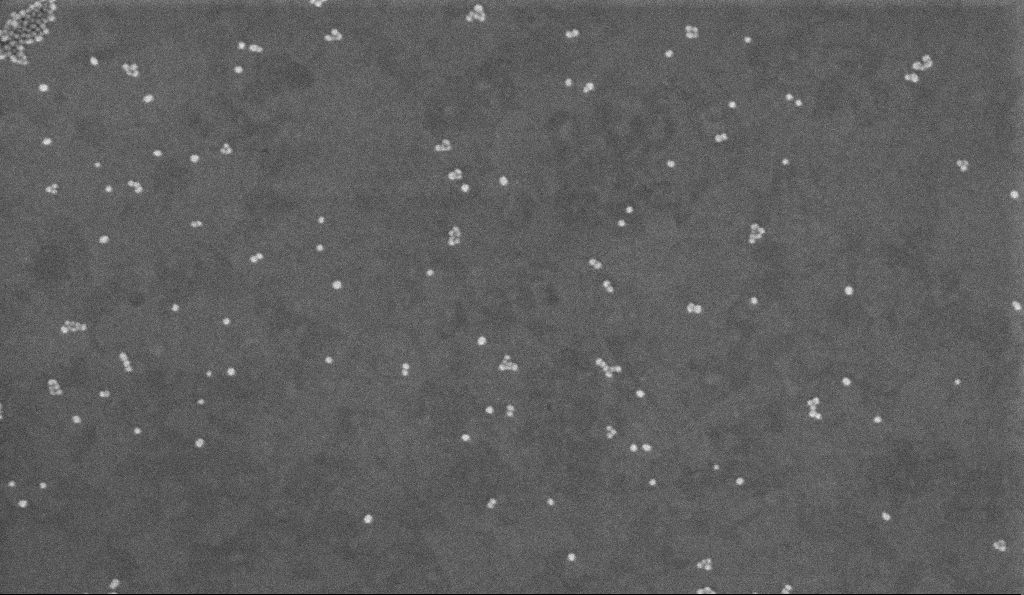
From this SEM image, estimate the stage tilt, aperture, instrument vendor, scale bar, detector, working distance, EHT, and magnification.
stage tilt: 0°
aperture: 30 µm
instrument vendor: Zeiss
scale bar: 200 nm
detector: InLens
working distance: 3.4 mm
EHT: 2 kV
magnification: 100 K X